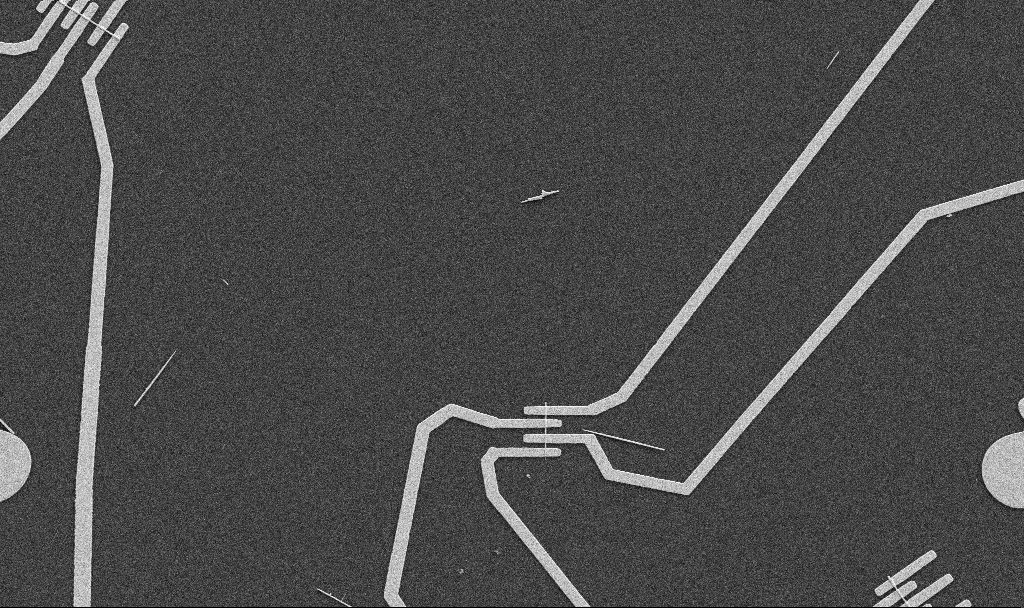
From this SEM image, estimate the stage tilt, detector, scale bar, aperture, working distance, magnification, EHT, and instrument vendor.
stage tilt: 0°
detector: SE2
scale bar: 10000 nm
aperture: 30 µm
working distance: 10.7 mm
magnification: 5 K X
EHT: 5 kV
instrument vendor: Zeiss